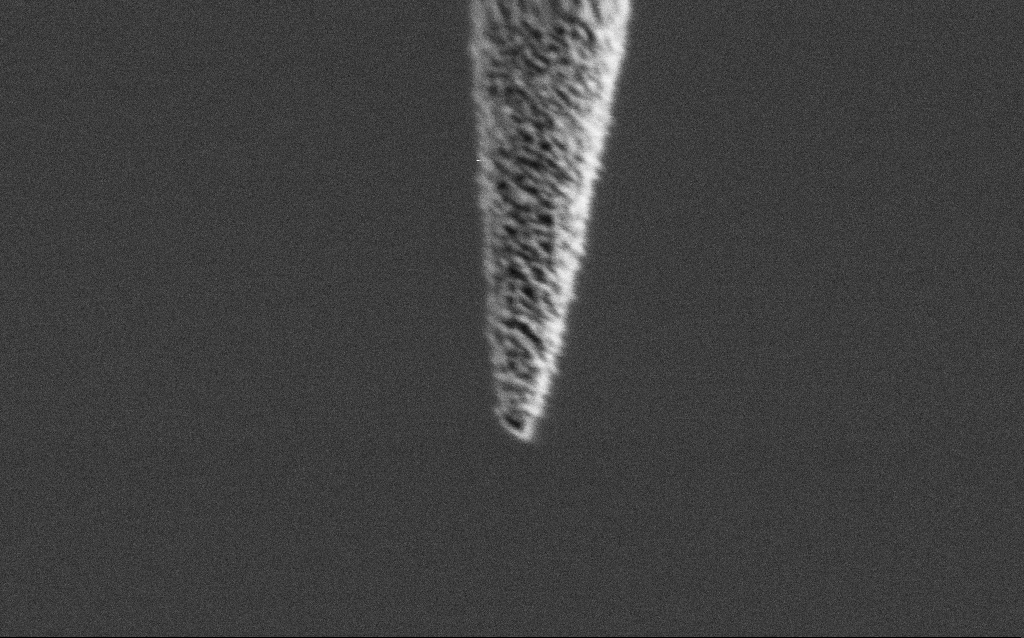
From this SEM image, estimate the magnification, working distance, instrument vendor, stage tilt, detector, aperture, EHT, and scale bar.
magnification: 100 K X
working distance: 6.7 mm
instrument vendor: Zeiss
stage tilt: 45°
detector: SE2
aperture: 30 µm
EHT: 1 kV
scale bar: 200 nm